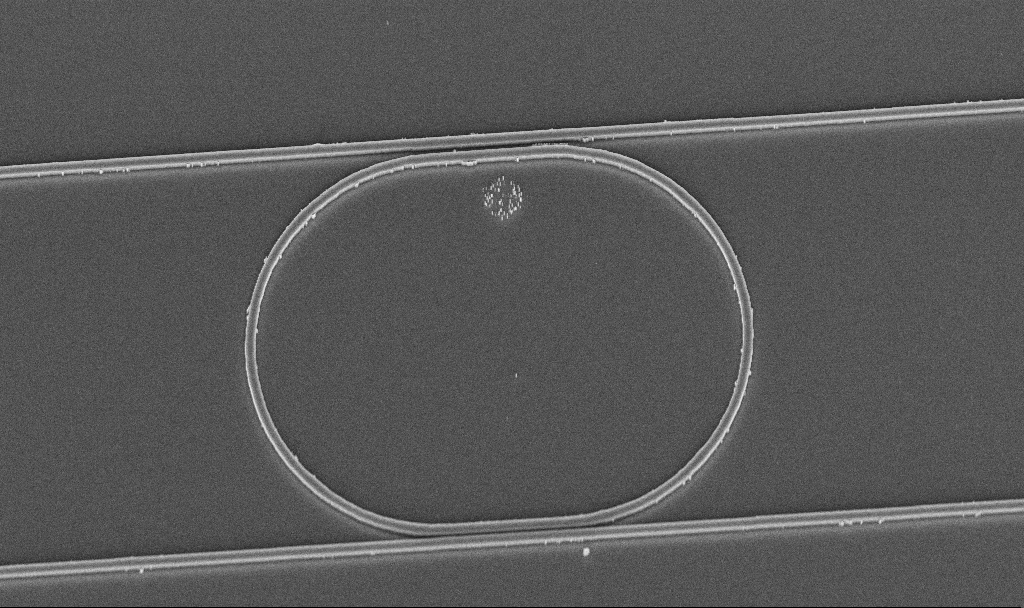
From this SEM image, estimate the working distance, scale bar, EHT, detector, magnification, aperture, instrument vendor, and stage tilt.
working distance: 9.8 mm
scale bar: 10000 nm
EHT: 5 kV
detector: InLens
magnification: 7.16 K X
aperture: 30 µm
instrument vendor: Zeiss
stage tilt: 45°